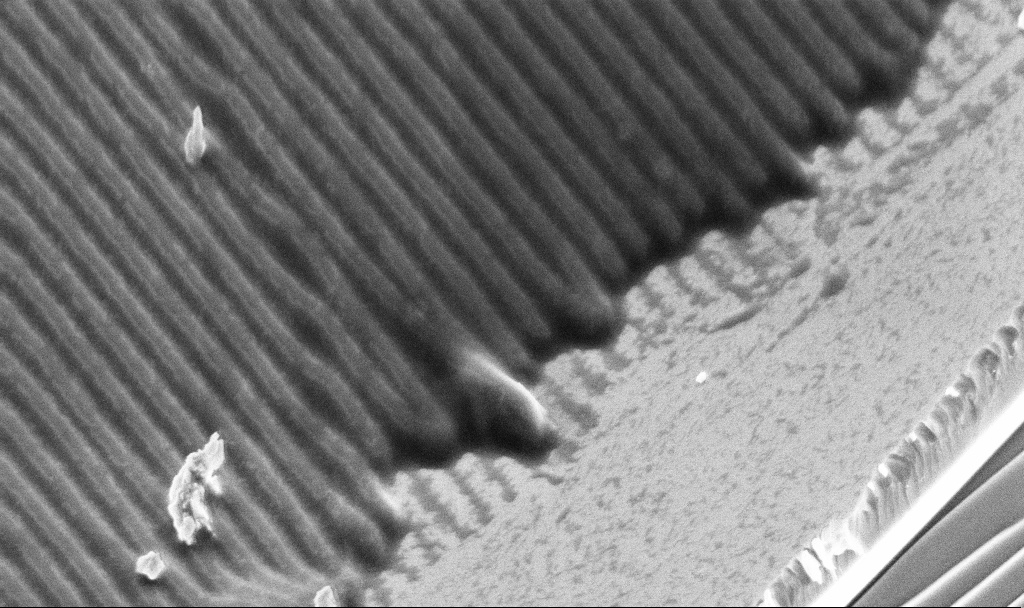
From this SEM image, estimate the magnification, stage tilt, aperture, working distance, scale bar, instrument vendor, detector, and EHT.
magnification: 25 K X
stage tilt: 45°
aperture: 30 µm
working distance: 2 mm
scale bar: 1000 nm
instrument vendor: Zeiss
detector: InLens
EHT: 3 kV